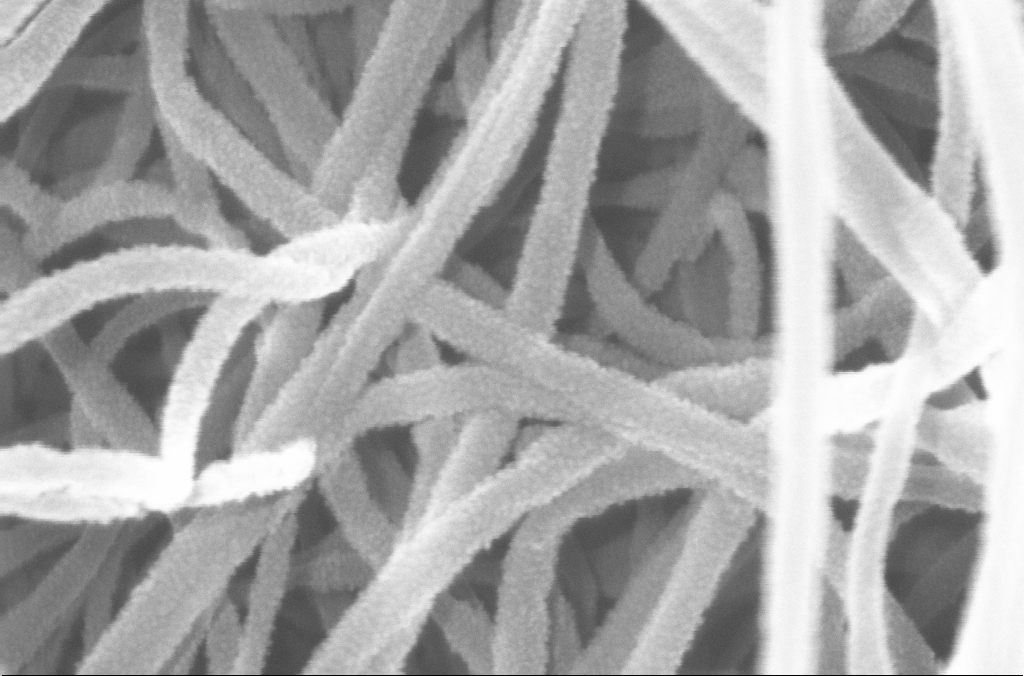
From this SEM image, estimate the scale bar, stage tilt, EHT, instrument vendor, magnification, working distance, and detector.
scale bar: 100 nm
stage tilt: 0°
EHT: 20 kV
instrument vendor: Zeiss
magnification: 400 K X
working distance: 4.2 mm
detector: InLens